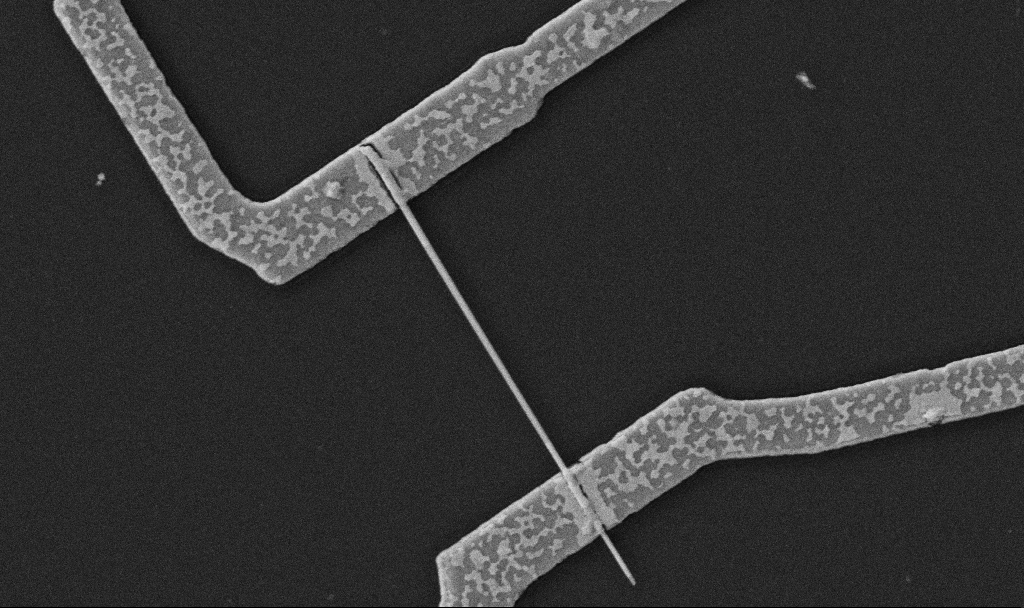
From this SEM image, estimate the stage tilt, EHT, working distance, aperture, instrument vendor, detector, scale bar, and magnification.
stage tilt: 0°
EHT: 5 kV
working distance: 10.7 mm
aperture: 30 µm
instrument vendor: Zeiss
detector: SE2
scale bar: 1000 nm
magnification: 30 K X